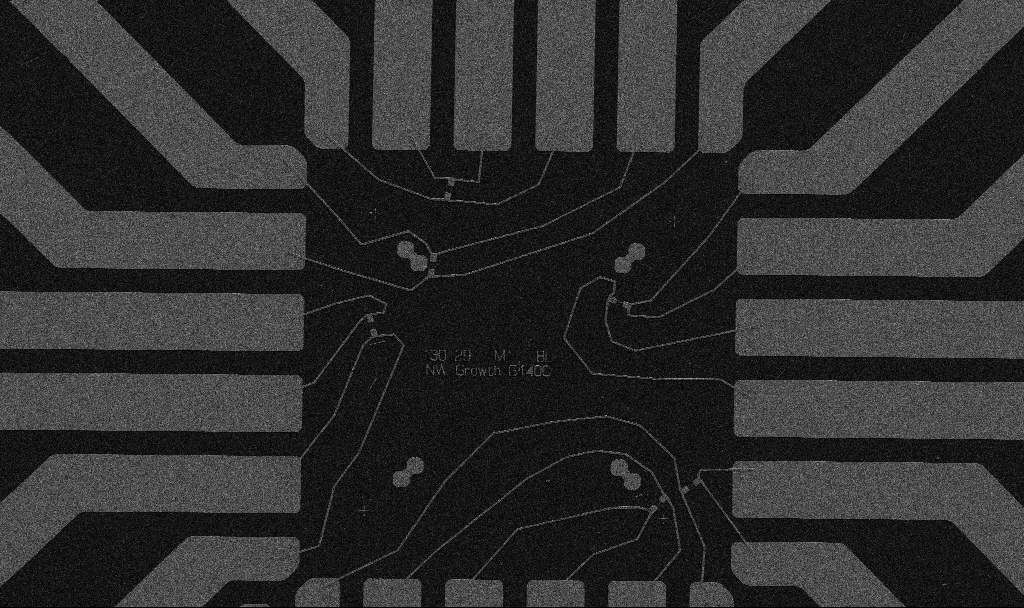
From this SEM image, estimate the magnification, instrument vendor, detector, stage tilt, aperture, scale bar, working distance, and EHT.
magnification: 1 K X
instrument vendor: Zeiss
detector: SE2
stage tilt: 0°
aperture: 30 µm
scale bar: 20000 nm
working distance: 8.7 mm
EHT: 5 kV